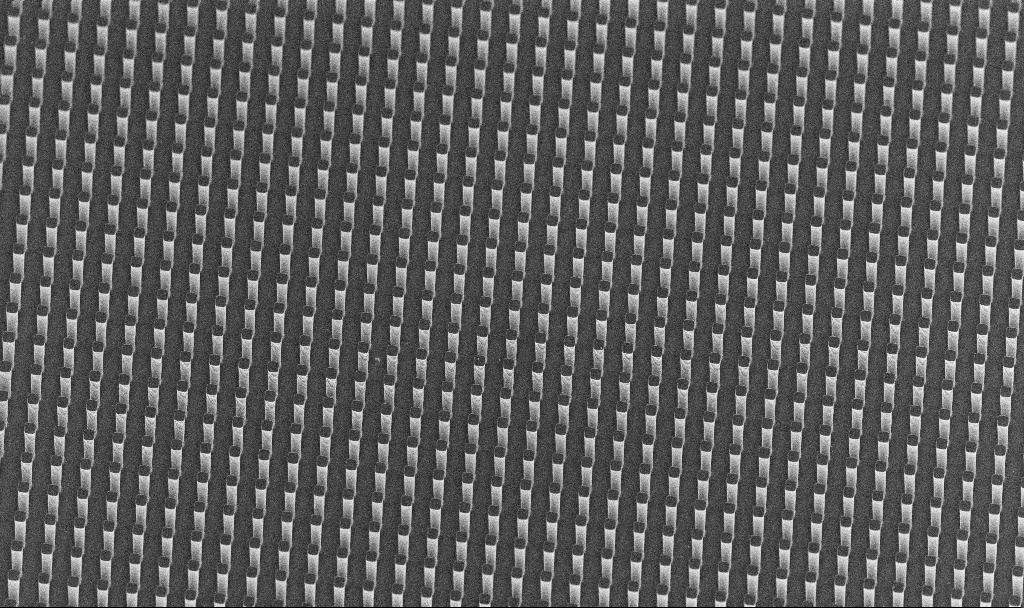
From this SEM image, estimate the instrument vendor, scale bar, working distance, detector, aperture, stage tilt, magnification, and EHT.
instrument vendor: Zeiss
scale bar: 20000 nm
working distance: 10.1 mm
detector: InLens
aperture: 30 µm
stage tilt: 45°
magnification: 0.897 K X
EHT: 5 kV